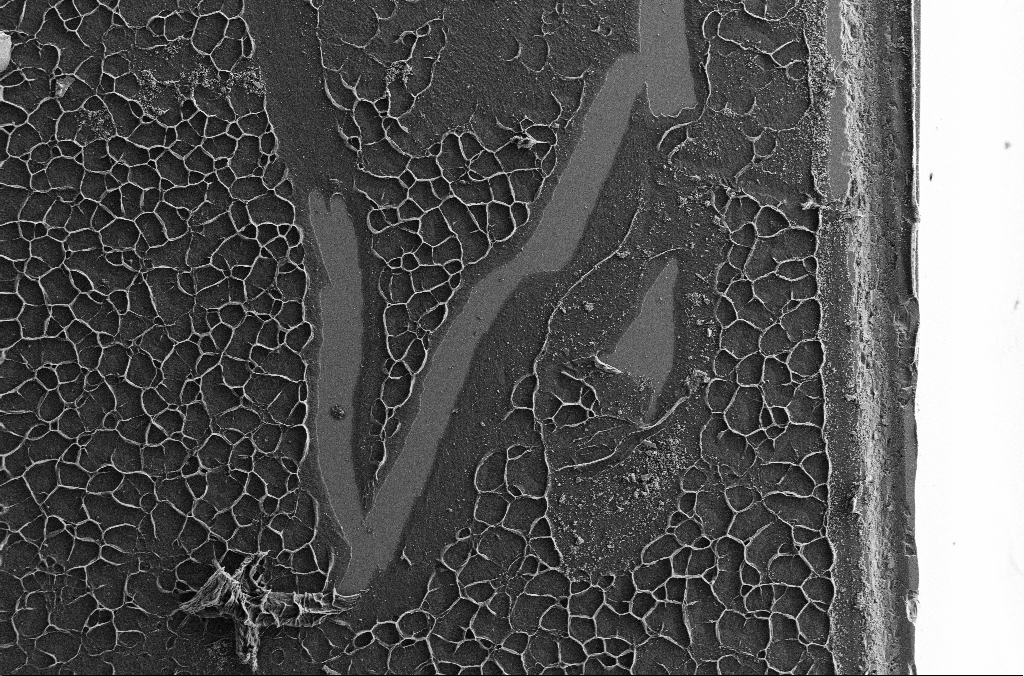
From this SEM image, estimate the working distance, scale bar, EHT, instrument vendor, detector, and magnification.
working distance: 3 mm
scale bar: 100000 nm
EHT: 7 kV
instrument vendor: Zeiss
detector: SE2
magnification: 0.2 K X